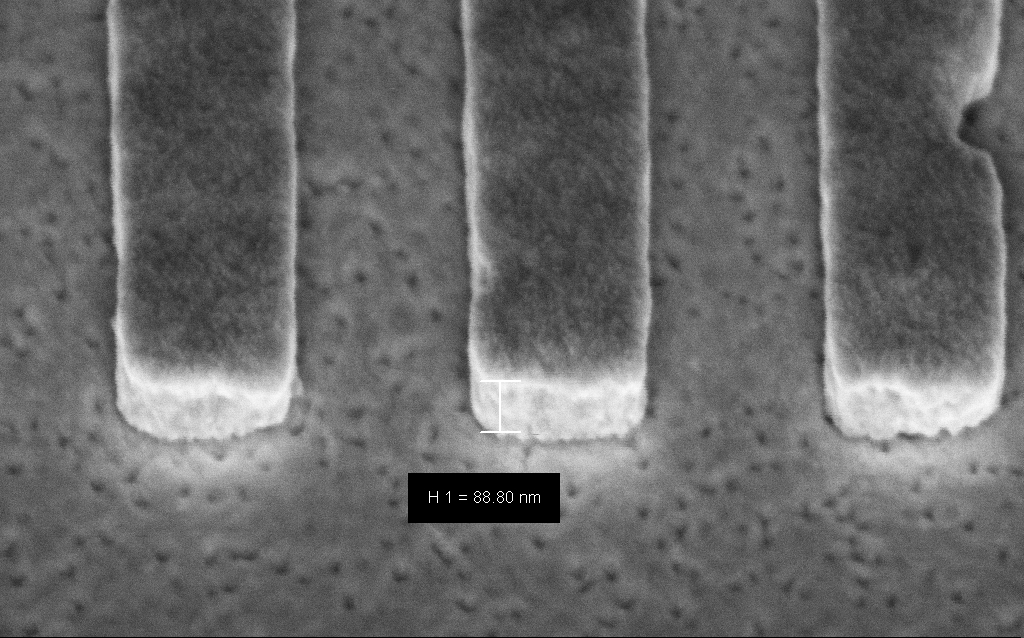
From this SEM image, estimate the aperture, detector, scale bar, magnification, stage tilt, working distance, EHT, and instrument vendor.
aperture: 30 µm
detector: InLens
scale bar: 200 nm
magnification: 210.88 K X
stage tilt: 45°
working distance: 6.6 mm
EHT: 3 kV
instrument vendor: Zeiss